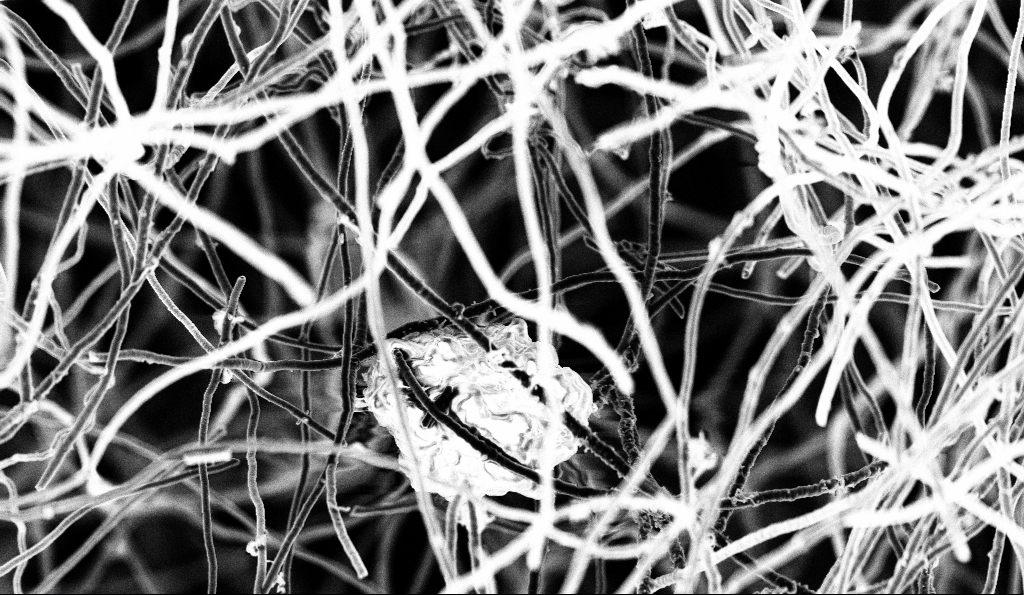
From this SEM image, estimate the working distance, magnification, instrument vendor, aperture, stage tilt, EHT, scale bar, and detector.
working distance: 5.5 mm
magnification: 5 K X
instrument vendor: Zeiss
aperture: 30 µm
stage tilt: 0°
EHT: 3 kV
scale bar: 10000 nm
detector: InLens